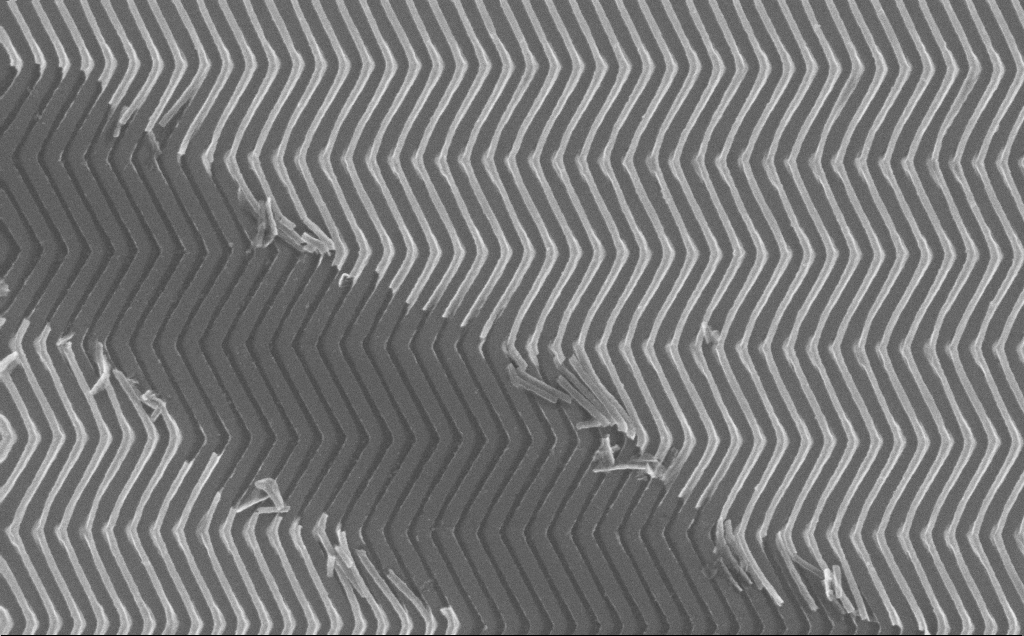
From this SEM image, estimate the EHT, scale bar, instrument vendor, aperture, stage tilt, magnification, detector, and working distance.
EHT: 10 kV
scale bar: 1000 nm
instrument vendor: Zeiss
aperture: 30 µm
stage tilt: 0°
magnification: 34.44 K X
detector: InLens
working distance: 7 mm